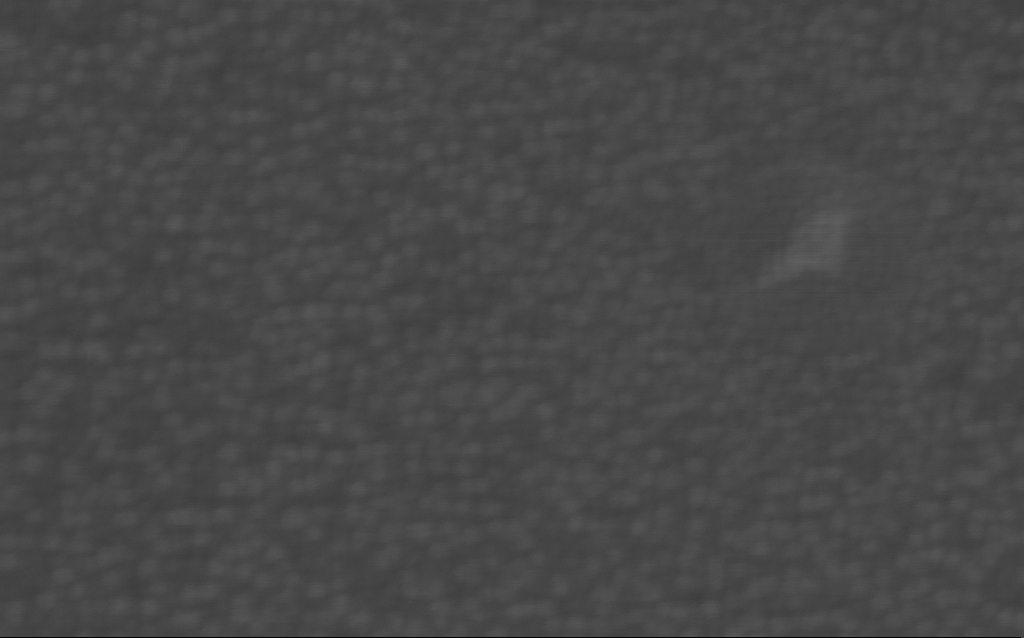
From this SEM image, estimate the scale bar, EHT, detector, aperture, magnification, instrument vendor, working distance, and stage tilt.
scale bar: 100 nm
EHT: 3 kV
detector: InLens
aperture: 30 µm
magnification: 468.2 K X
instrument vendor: Zeiss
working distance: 3 mm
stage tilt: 0°